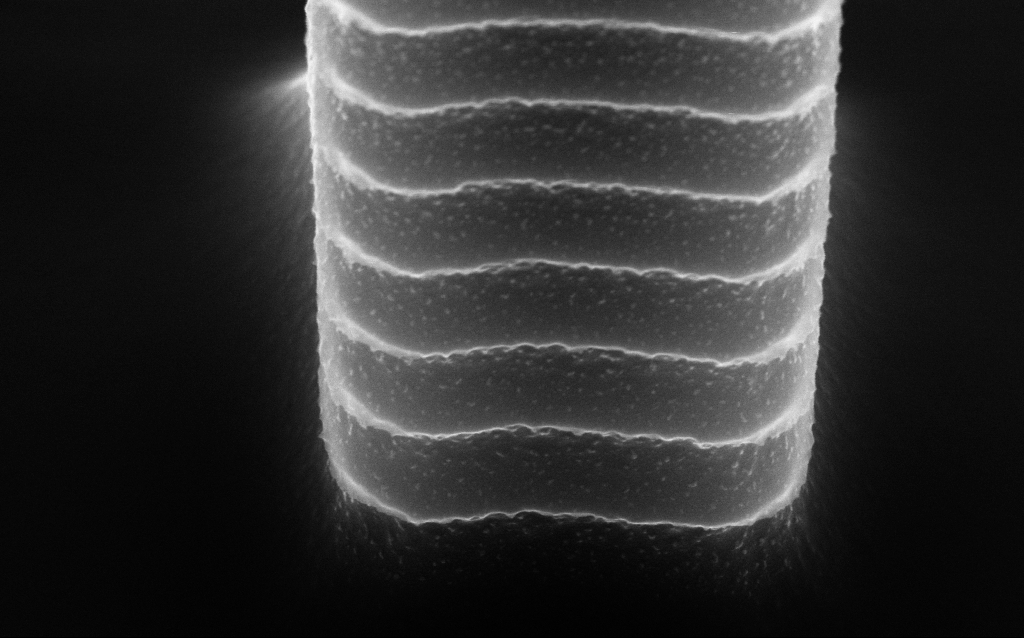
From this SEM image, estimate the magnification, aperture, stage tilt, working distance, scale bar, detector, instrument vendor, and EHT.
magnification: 125.43 K X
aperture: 30 µm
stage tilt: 45°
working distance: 4.8 mm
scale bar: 200 nm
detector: InLens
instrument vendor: Zeiss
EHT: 10 kV